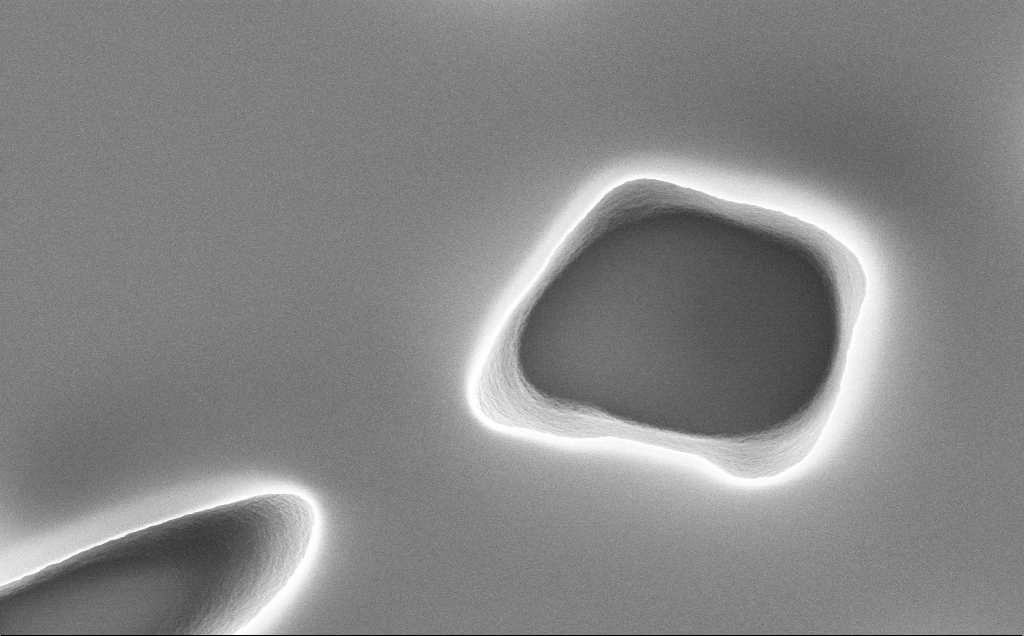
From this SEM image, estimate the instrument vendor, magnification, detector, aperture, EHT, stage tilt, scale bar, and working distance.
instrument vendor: Zeiss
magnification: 36.1 K X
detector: InLens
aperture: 30 µm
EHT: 10 kV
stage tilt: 0°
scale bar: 2000 nm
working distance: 12 mm